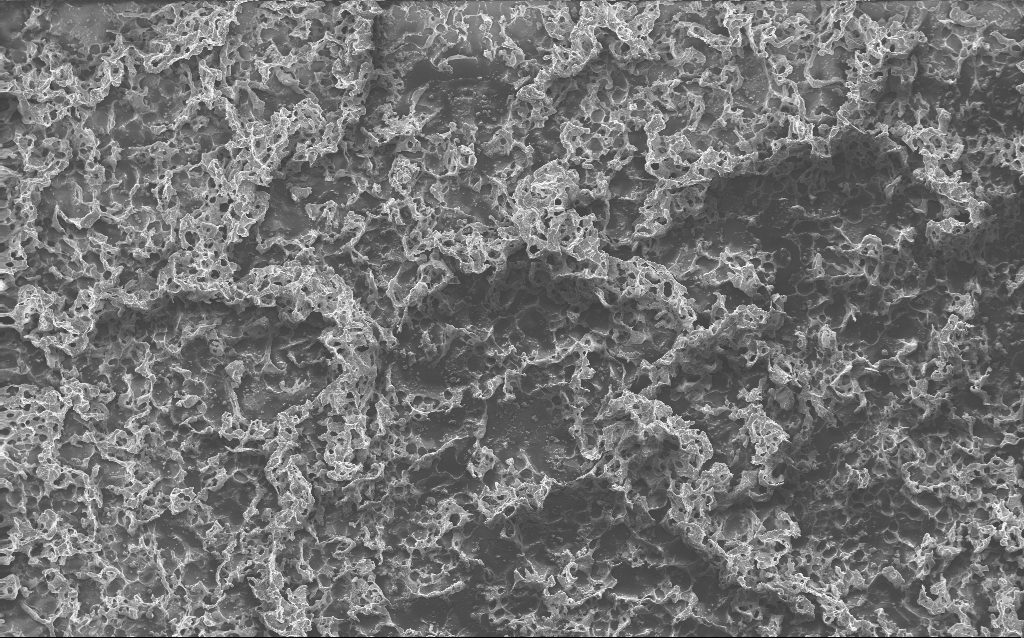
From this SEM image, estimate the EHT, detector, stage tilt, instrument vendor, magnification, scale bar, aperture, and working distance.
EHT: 10 kV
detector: InLens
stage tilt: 0°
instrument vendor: Zeiss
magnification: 0.235 K X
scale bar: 100000 nm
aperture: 30 µm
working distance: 2.7 mm